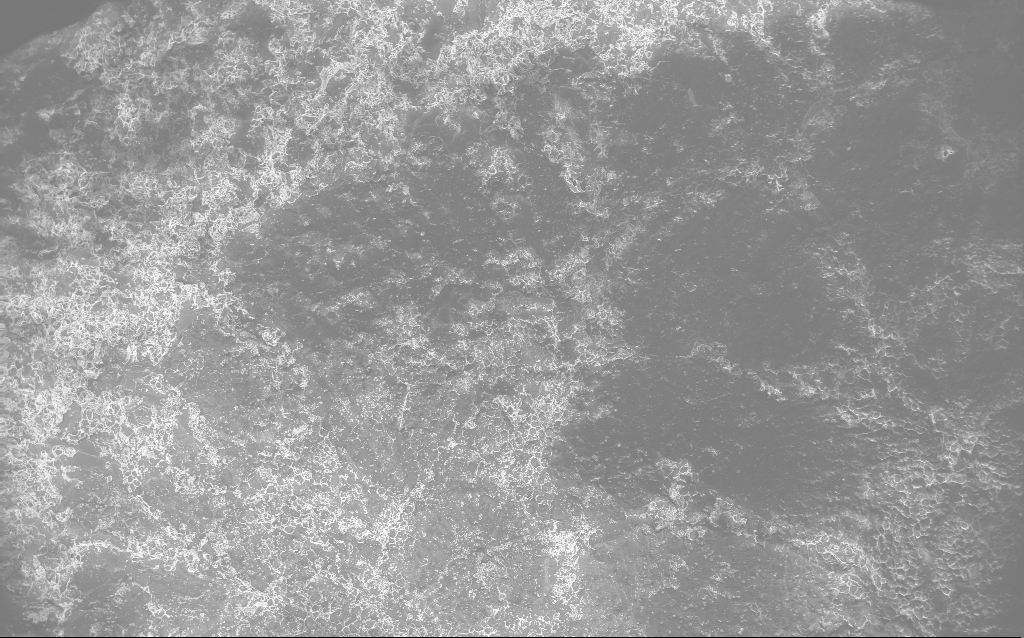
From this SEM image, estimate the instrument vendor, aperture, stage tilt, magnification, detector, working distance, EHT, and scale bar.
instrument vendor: Zeiss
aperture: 30 µm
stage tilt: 0°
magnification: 0.122 K X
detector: InLens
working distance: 2.8 mm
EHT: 10 kV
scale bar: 100000 nm